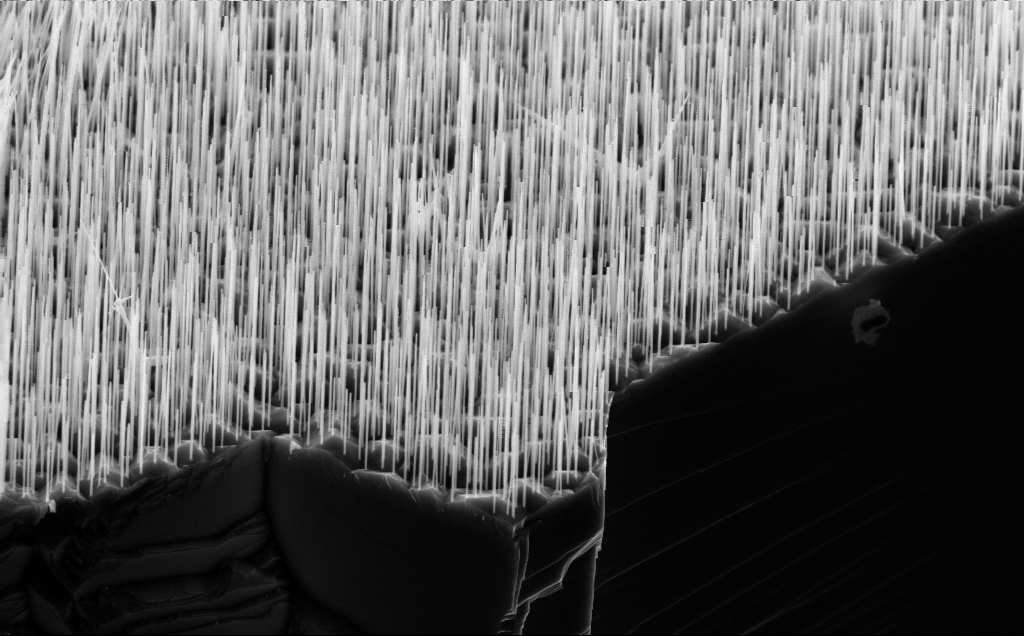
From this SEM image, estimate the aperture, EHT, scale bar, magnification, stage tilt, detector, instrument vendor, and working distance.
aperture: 30 µm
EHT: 10 kV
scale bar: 2000 nm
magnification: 18.66 K X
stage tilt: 45°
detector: InLens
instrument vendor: Zeiss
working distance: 5 mm